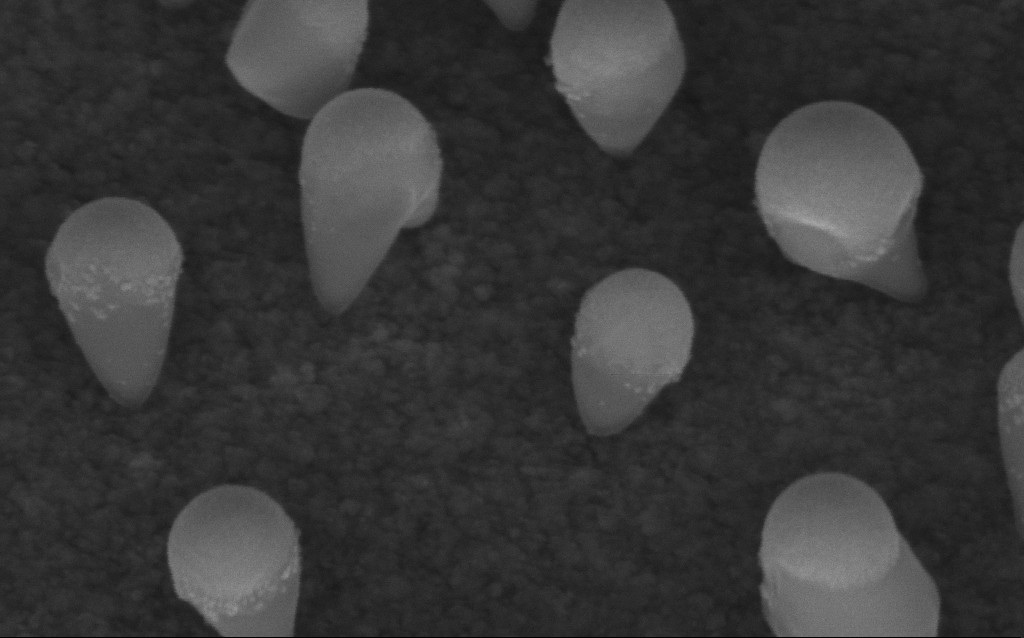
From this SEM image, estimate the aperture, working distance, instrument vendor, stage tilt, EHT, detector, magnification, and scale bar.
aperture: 30 µm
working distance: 6.4 mm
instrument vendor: Zeiss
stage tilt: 45°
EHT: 5 kV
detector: InLens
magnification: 290.69 K X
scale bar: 100 nm